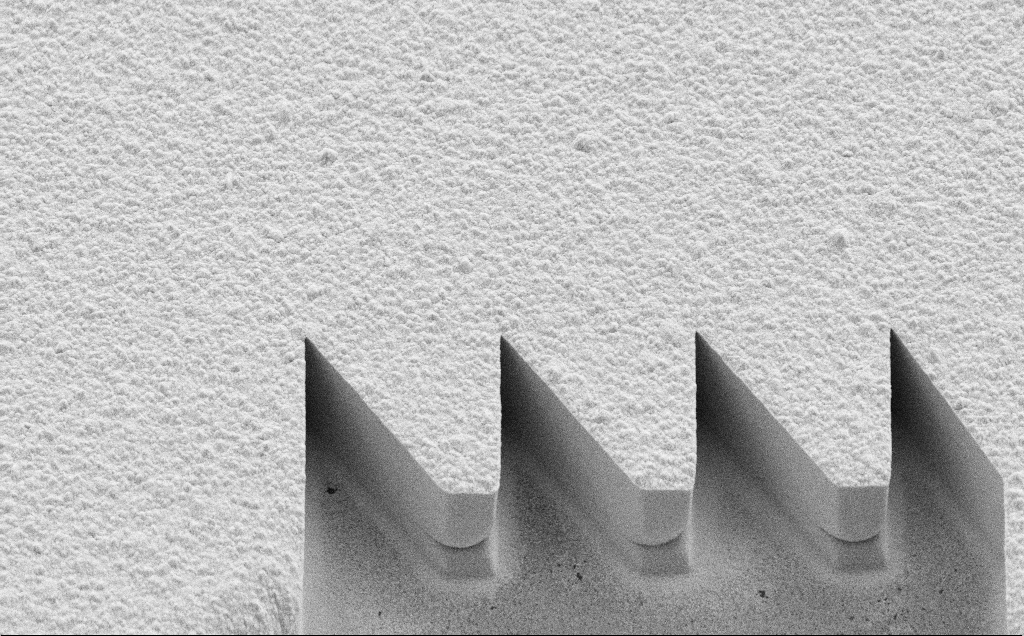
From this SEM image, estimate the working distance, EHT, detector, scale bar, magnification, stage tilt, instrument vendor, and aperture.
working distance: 7 mm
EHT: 10 kV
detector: SE2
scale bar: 10000 nm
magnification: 6.26 K X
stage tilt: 45°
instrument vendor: Zeiss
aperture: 30 µm